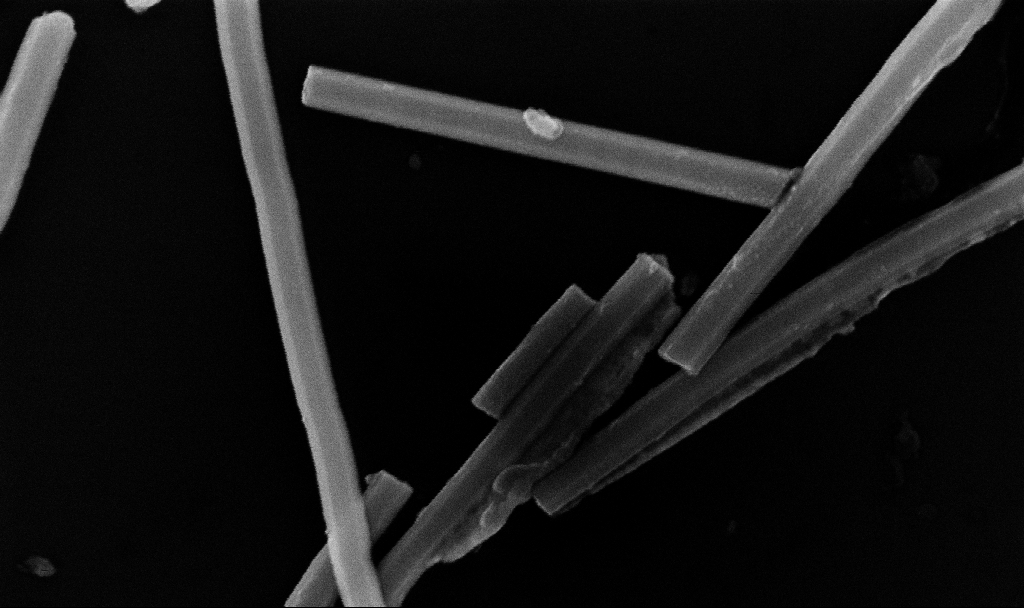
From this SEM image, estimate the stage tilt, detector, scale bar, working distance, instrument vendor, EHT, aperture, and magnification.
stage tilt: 0°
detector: InLens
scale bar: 200 nm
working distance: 10.7 mm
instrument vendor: Zeiss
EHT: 10 kV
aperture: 30 µm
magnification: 145 K X